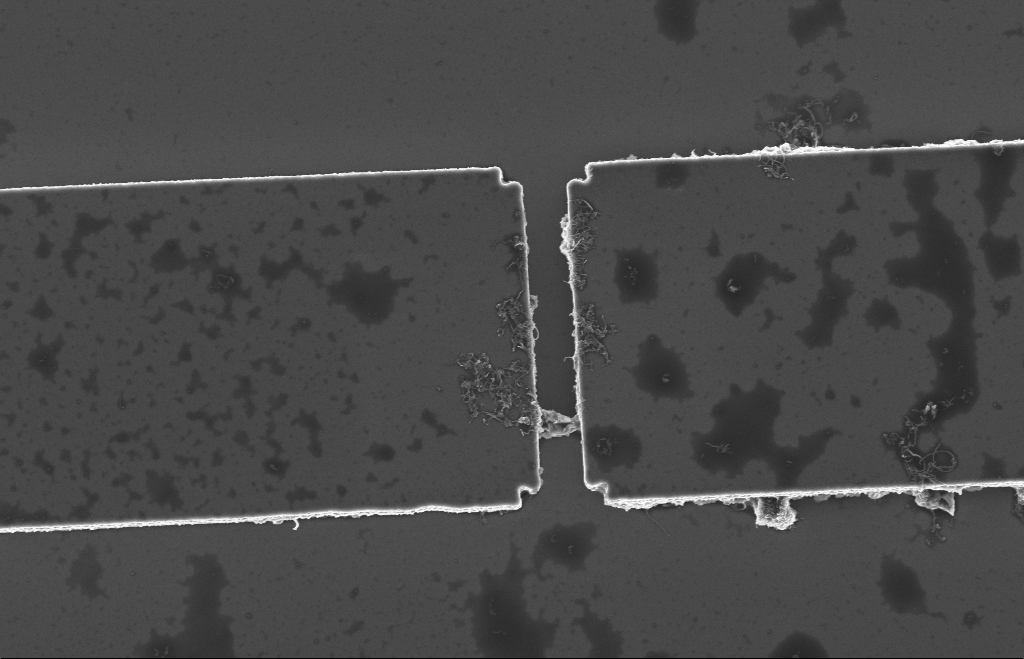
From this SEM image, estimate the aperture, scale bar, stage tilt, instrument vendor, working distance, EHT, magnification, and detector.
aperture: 20 µm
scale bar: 2000 nm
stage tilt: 0.4°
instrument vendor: Zeiss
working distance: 9 mm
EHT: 5 kV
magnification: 6.68 K X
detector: InLens